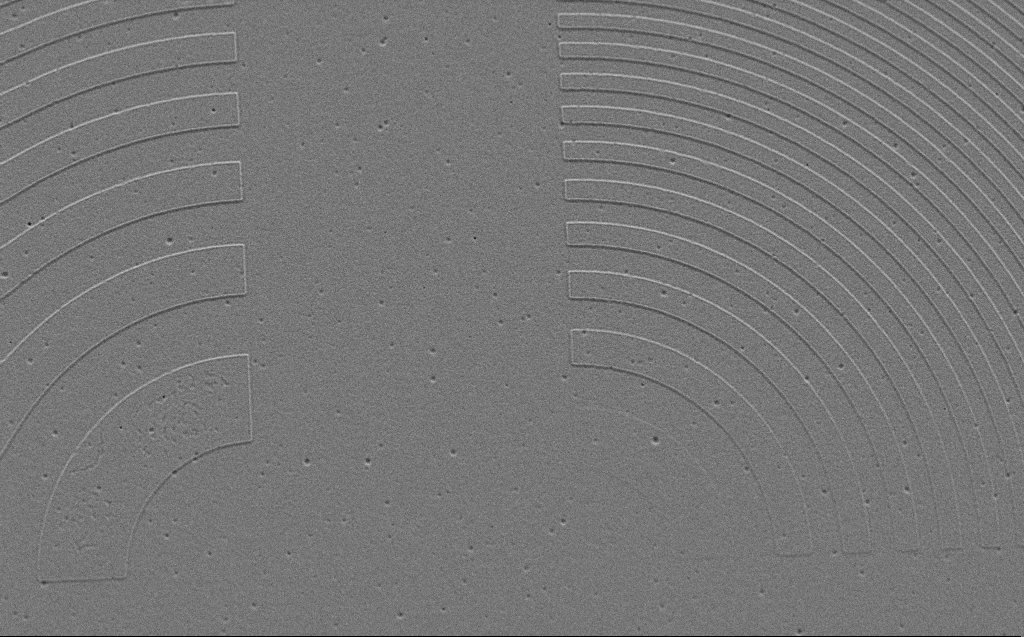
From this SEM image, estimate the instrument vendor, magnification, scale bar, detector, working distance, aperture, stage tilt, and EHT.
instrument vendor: Zeiss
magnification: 11.96 K X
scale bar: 2000 nm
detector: SE2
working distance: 4 mm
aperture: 30 µm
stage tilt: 29.8°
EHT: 2 kV